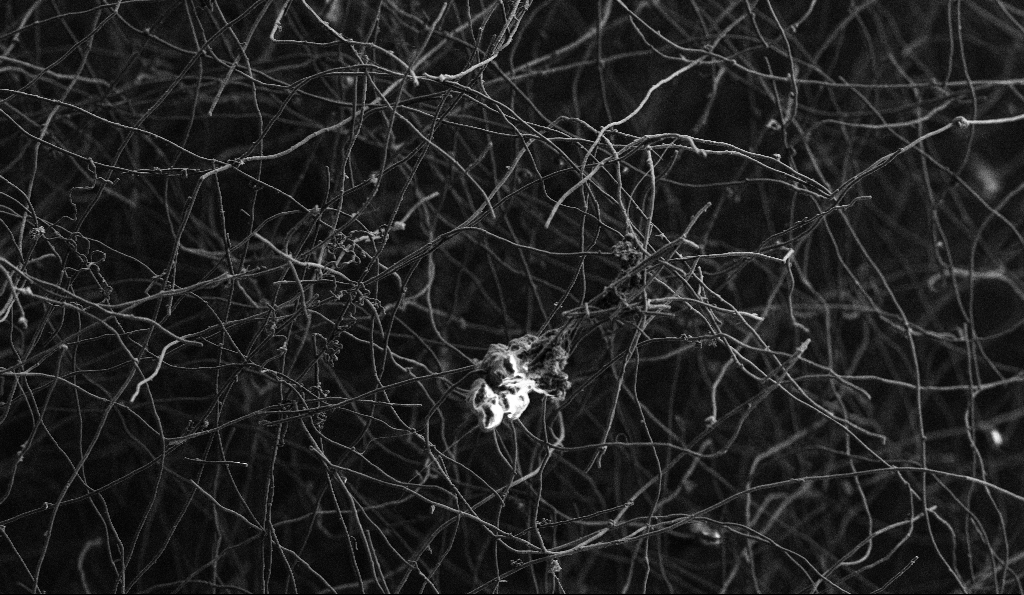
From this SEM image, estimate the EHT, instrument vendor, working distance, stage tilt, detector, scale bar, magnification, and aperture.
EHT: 3 kV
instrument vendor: Zeiss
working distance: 5.5 mm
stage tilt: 0°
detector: SE2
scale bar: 10000 nm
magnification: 2 K X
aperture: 30 µm